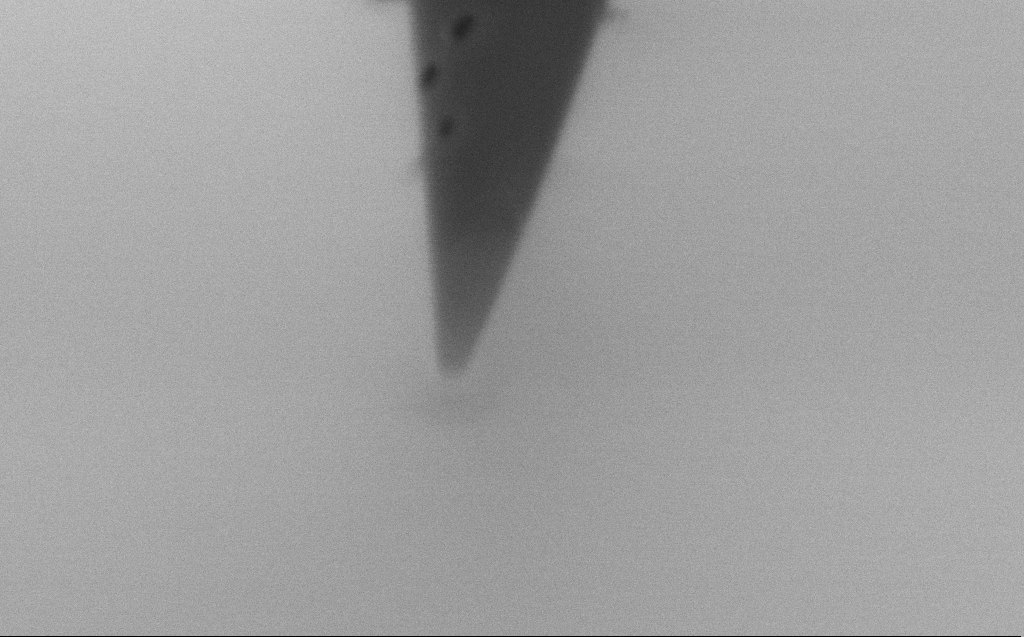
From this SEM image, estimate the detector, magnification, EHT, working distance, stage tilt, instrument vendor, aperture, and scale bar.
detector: InLens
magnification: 189.26 K X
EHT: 1.5 kV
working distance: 3 mm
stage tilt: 45.1°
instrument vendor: Zeiss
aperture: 20 µm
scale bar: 200 nm